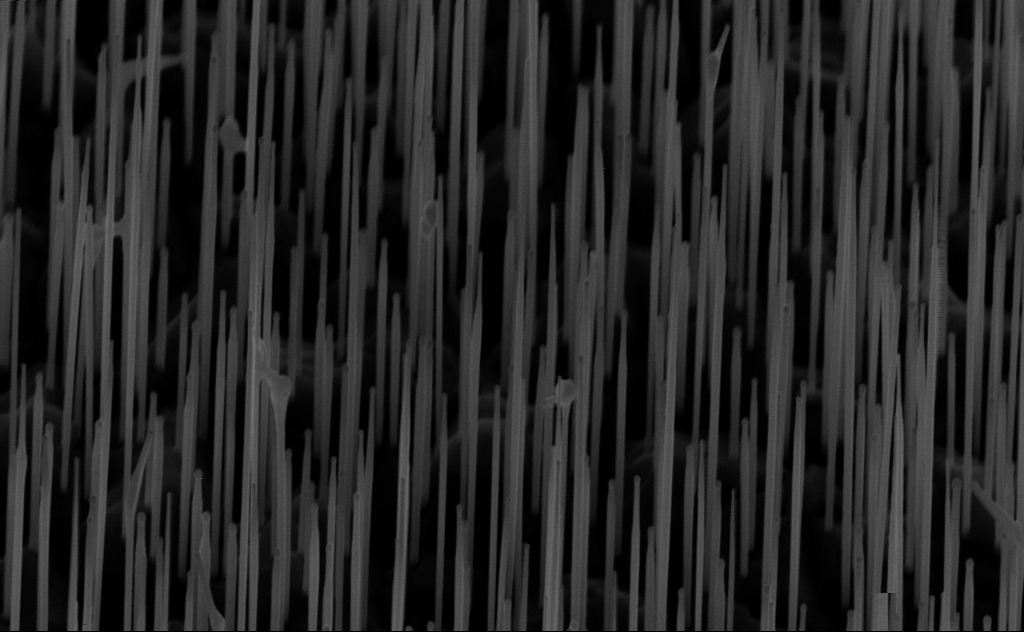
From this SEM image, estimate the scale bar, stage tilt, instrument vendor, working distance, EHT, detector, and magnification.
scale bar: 1000 nm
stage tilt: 45°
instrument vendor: Zeiss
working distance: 6 mm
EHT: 10 kV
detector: InLens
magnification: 40 K X